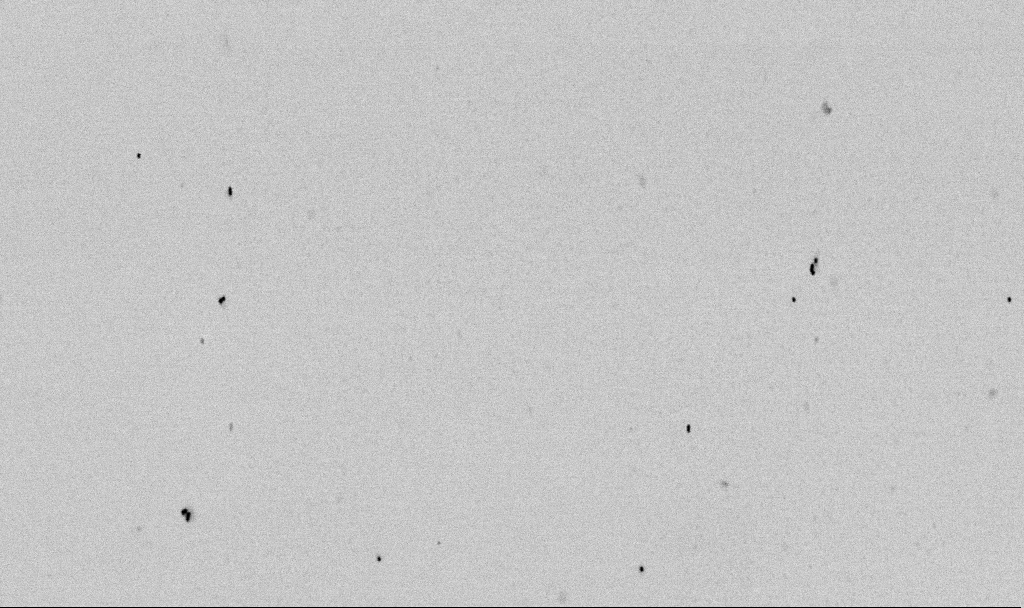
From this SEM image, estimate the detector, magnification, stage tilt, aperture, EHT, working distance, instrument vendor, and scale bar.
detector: SE2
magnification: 50 K X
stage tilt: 0°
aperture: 30 µm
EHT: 2 kV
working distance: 6.5 mm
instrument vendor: Zeiss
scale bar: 1000 nm